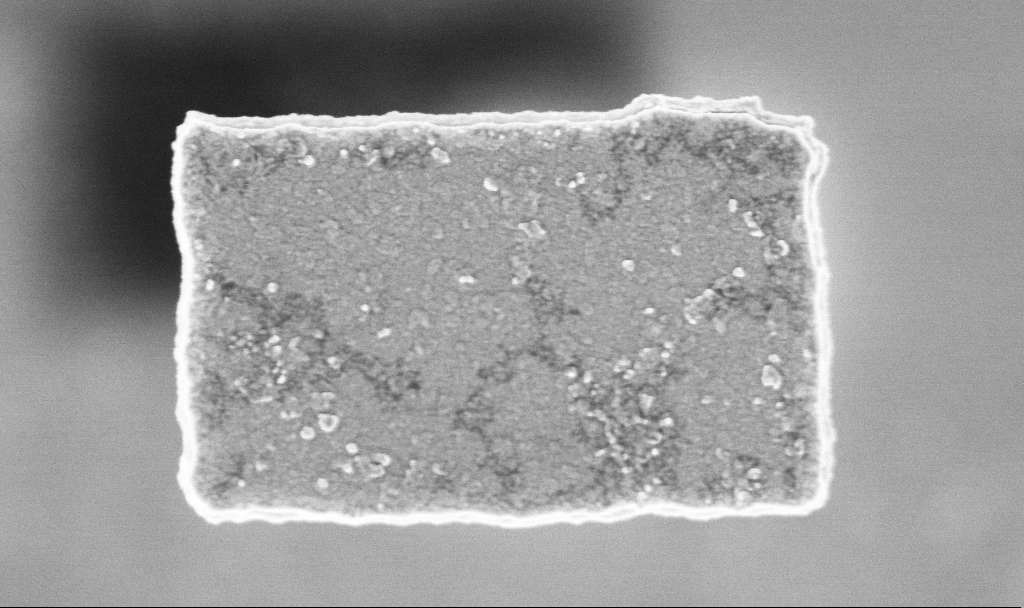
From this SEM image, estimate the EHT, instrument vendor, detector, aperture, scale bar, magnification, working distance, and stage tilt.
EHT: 3 kV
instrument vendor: Zeiss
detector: InLens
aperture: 30 µm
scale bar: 200 nm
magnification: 80.85 K X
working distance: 3.2 mm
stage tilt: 0°